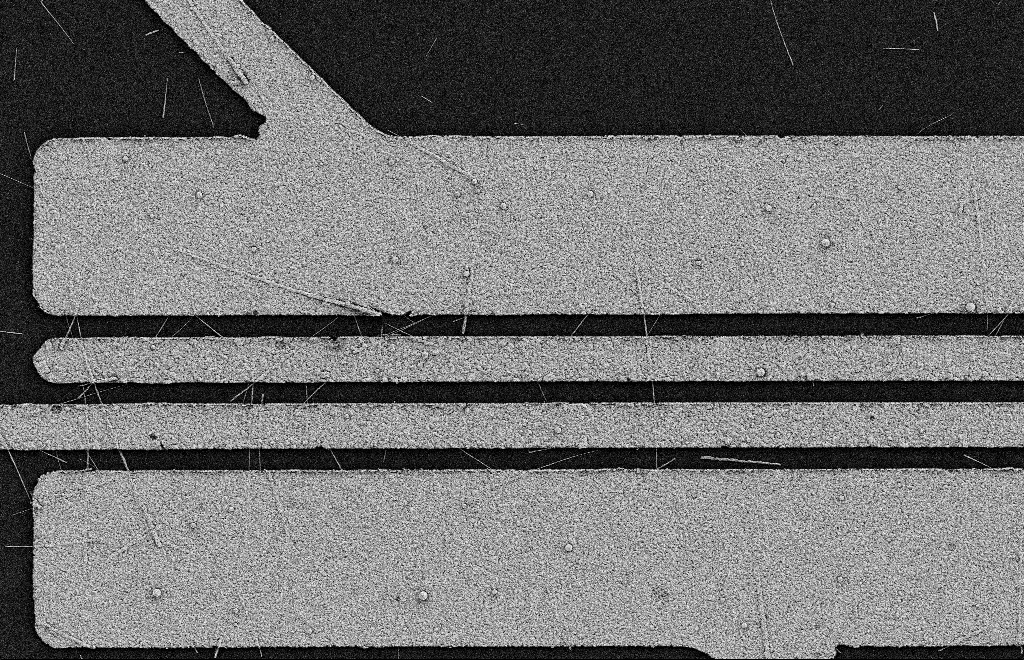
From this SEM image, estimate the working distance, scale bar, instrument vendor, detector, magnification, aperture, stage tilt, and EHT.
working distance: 11 mm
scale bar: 2000 nm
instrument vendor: Zeiss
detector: SE2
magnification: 6.1 K X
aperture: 20 µm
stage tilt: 0°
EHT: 2 kV